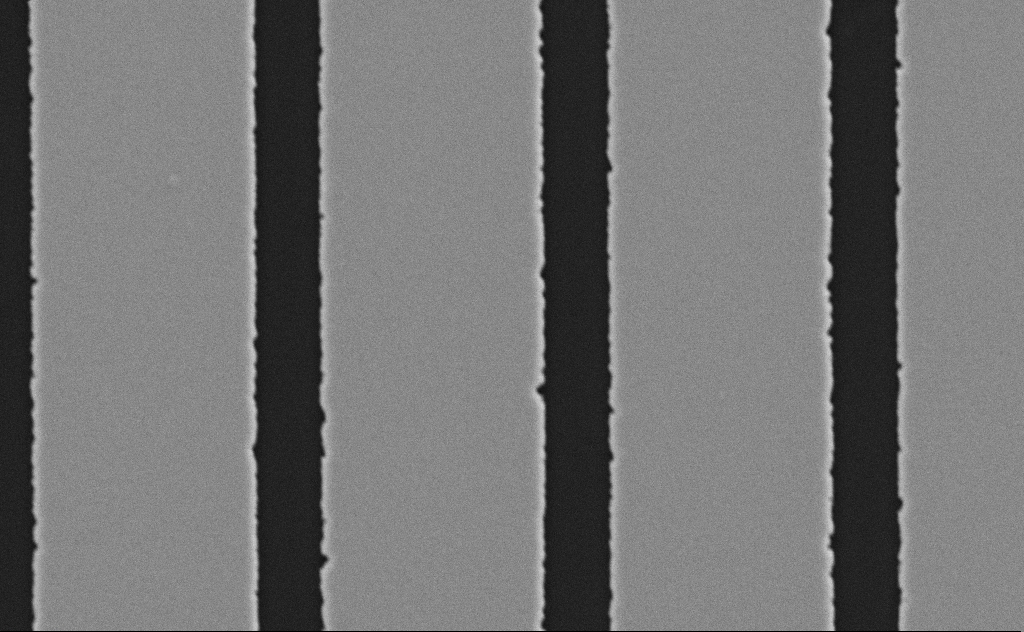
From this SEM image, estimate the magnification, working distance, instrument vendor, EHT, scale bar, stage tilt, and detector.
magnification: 26.43 K X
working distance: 8 mm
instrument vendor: Zeiss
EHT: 5 kV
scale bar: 2000 nm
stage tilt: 0°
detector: SE2